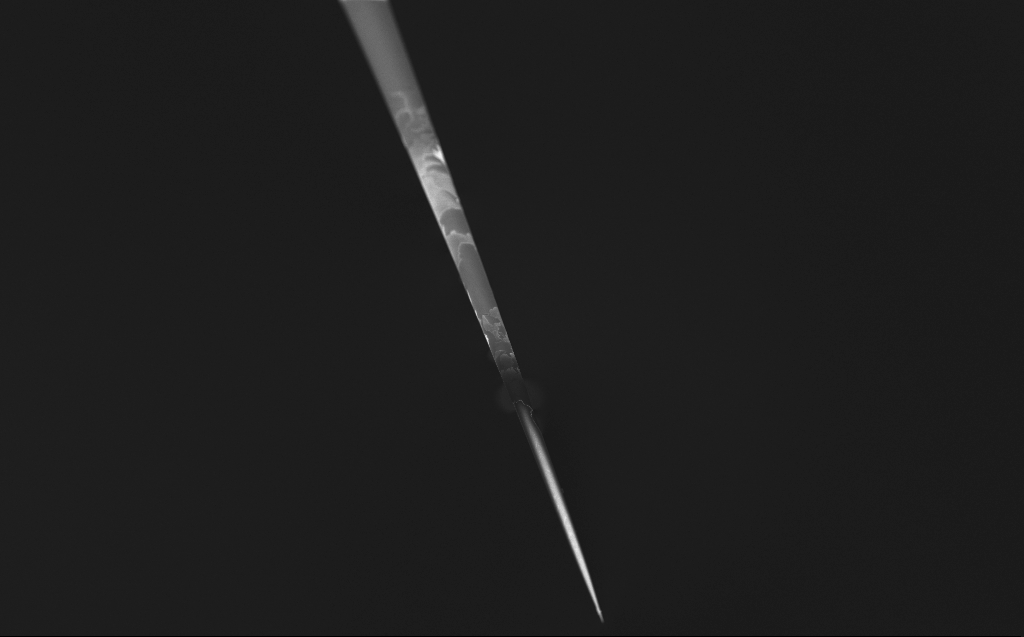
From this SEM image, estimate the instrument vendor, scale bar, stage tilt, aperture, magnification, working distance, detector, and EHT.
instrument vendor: Zeiss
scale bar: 100000 nm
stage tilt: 45°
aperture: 30 µm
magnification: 0.25 K X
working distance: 4 mm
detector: InLens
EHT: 1 kV